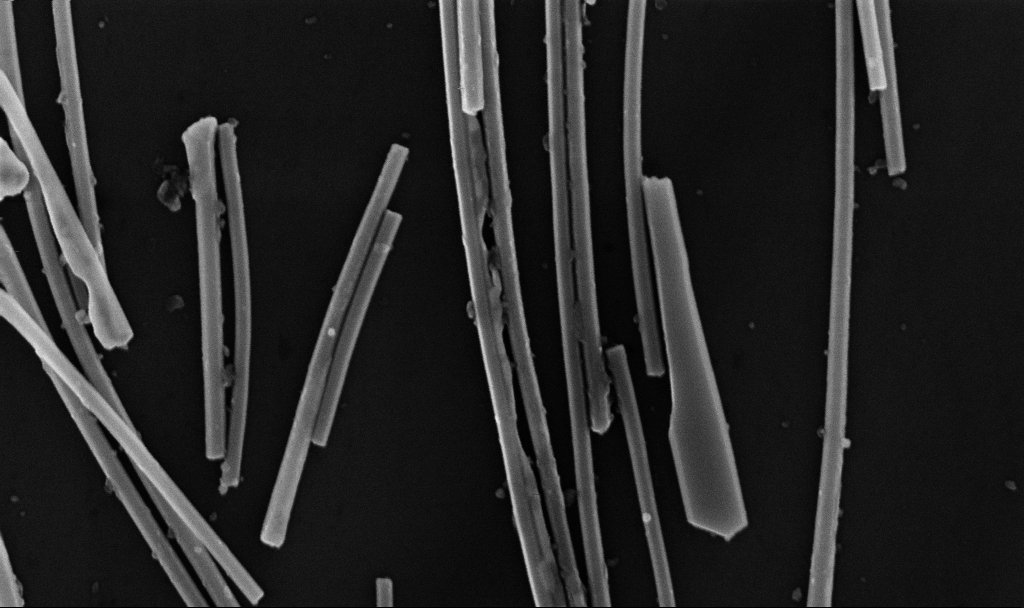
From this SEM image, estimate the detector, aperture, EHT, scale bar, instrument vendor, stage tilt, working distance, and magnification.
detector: InLens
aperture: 30 µm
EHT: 10 kV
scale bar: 1000 nm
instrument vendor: Zeiss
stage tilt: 0°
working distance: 6.7 mm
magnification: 64.39 K X